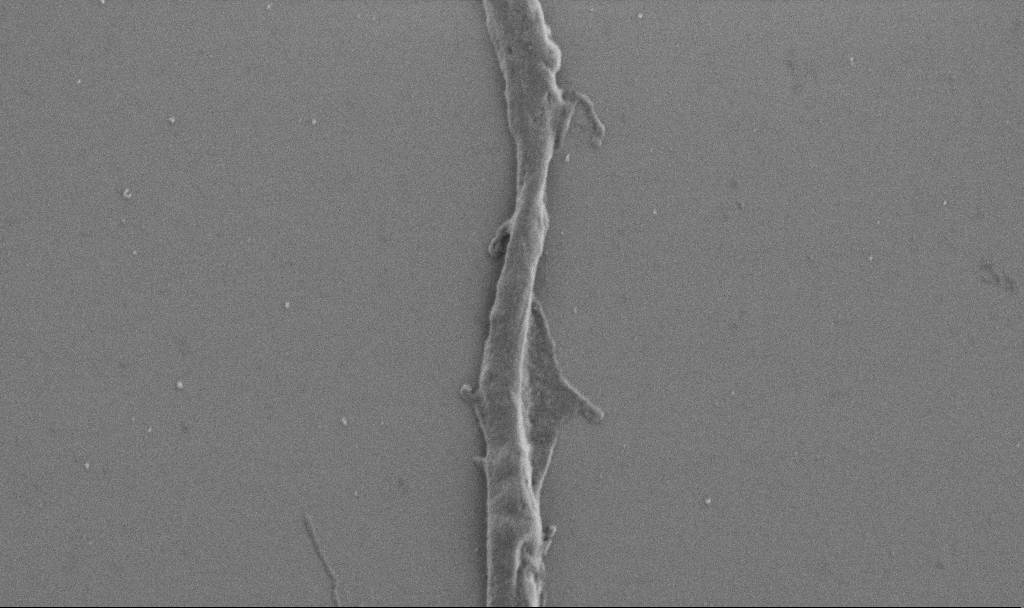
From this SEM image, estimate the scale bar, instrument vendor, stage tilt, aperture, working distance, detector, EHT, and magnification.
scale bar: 2000 nm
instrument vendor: Zeiss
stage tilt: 0°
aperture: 30 µm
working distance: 6.9 mm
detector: SE2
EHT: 1 kV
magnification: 20 K X